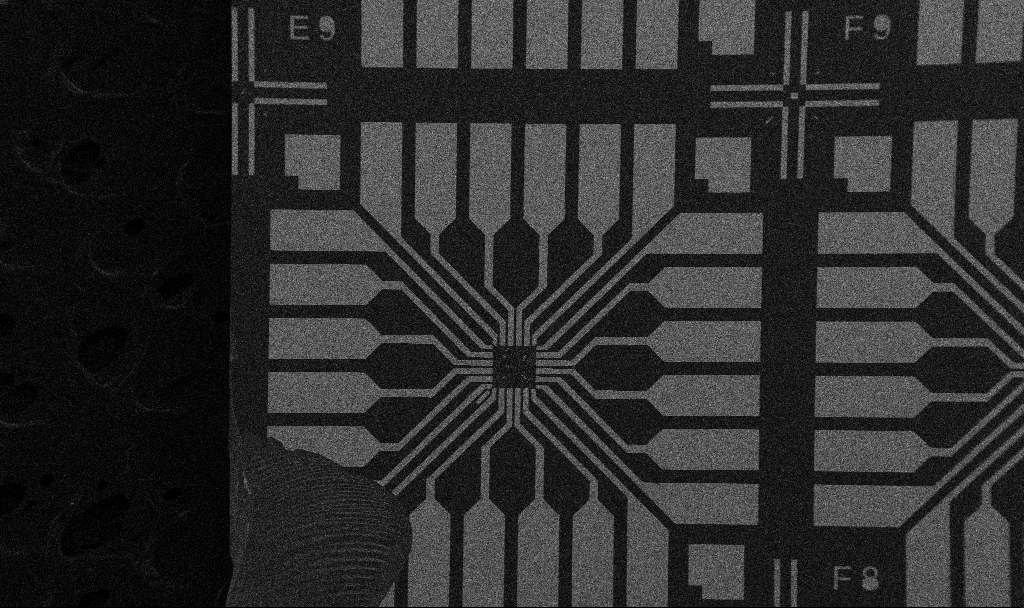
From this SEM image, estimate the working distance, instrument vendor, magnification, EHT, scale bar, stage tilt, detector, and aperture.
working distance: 10.7 mm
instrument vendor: Zeiss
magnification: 0.1 K X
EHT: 5 kV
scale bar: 200000 nm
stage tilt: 0°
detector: SE2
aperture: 30 µm